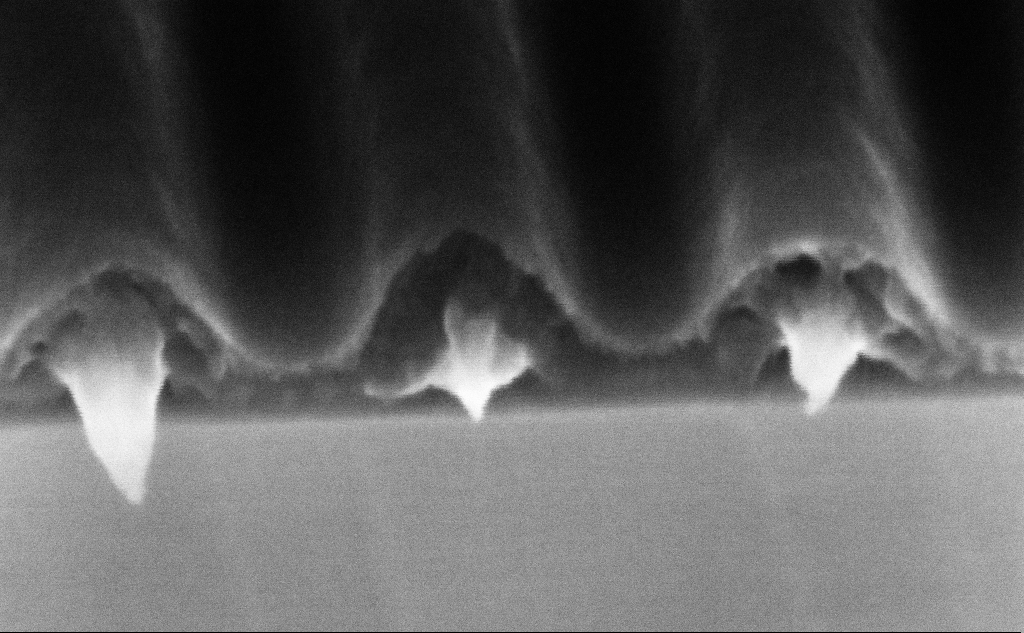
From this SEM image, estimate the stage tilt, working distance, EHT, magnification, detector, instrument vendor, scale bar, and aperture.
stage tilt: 45°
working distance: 7.4 mm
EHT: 5 kV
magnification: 433.13 K X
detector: InLens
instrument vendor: Zeiss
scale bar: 100 nm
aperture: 30 µm